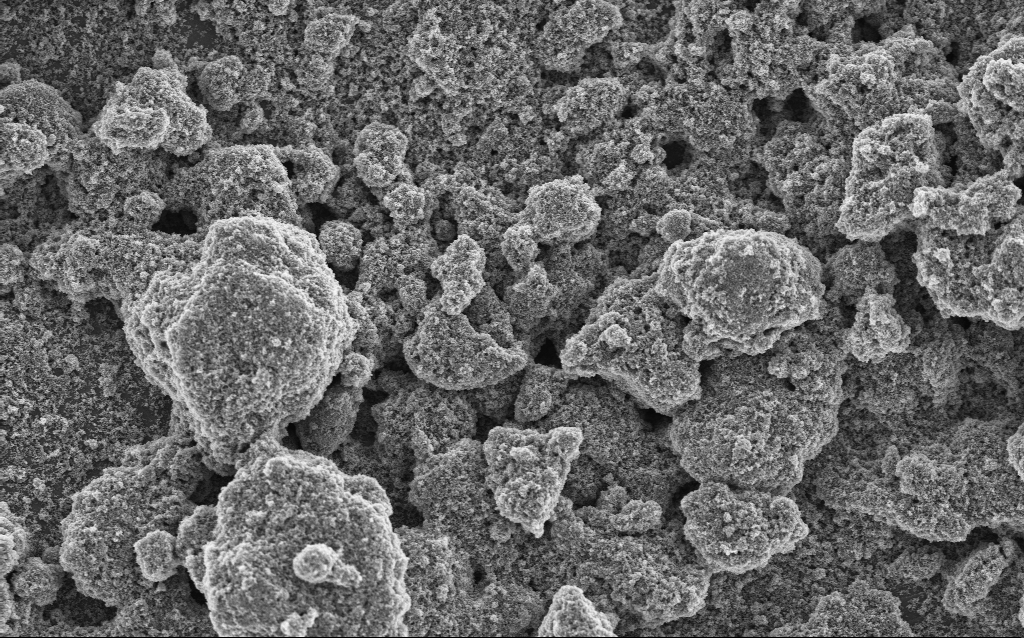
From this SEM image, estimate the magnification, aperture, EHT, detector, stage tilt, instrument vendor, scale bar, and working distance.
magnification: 6.41 K X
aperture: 30 µm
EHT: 5 kV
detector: InLens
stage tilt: -0°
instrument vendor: Zeiss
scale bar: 10000 nm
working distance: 4.2 mm